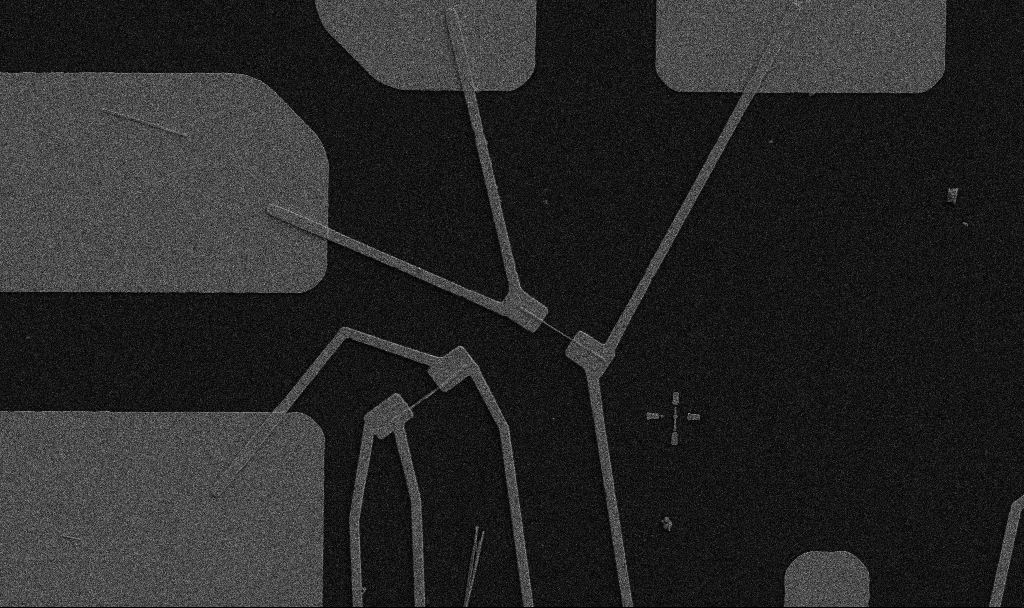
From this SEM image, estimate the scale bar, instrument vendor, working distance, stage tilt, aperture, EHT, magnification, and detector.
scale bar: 10000 nm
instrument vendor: Zeiss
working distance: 10.7 mm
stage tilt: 0°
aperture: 30 µm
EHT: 5 kV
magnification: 5 K X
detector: SE2